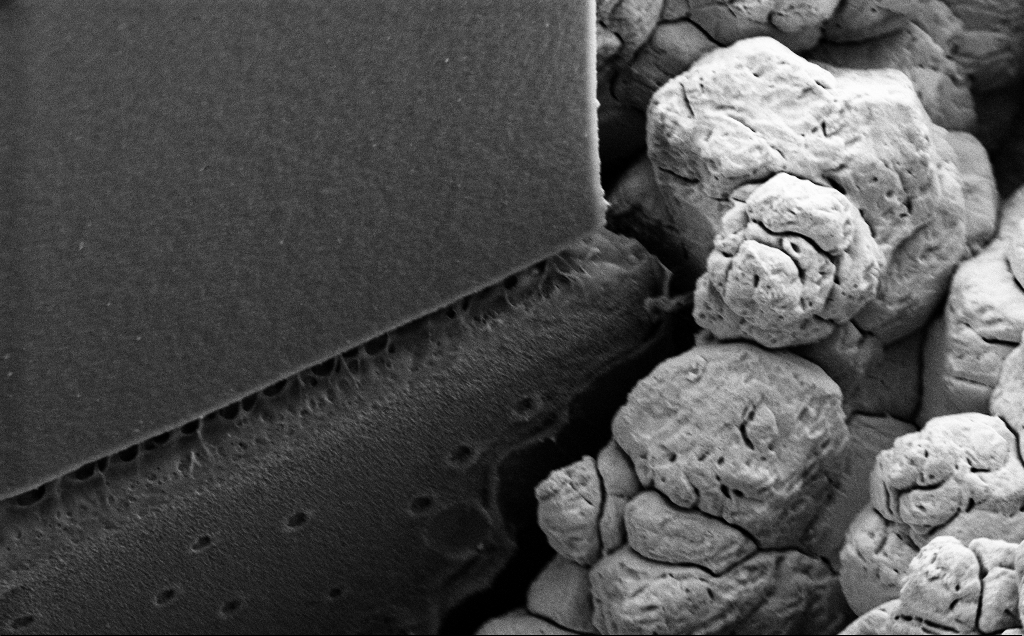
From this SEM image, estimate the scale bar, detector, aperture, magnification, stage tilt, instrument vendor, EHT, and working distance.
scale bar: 2000 nm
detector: SE2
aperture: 30 µm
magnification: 11.43 K X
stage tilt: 35°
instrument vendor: Zeiss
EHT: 5 kV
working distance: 9 mm